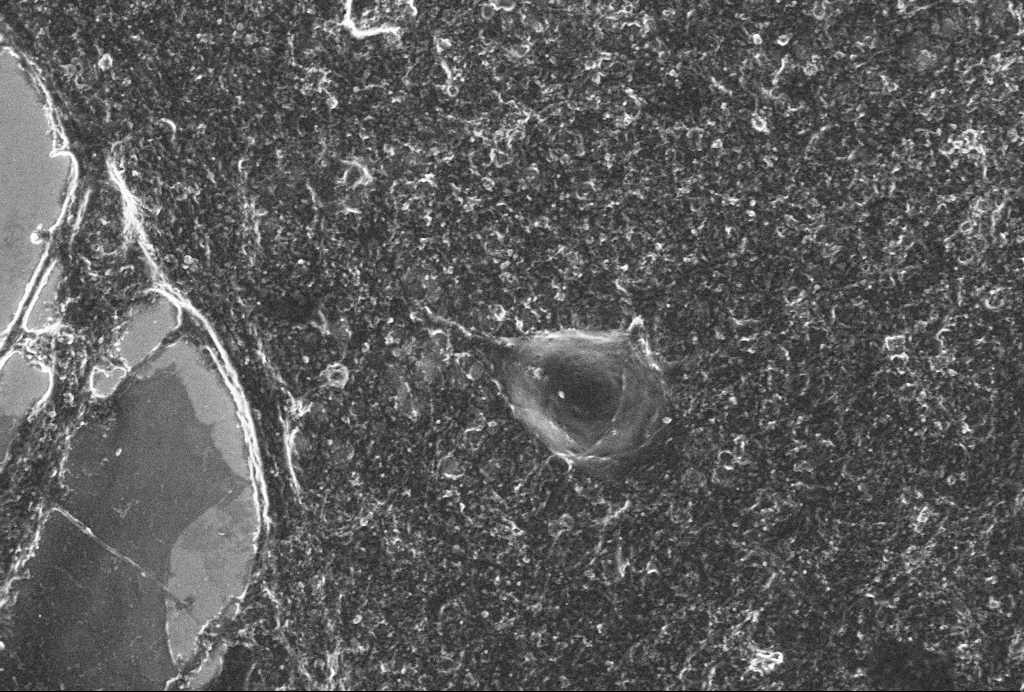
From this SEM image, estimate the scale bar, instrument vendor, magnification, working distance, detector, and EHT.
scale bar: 10000 nm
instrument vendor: Zeiss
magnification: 4 K X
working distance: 6 mm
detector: InLens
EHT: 4 kV